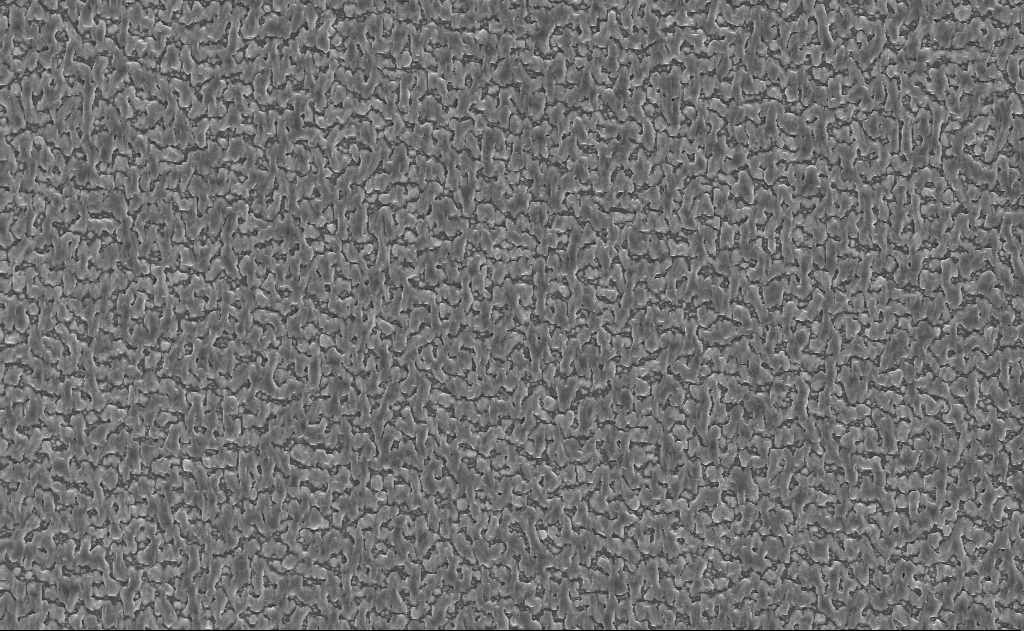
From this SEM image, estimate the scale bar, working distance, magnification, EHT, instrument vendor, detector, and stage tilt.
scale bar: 10000 nm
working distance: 14 mm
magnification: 5 K X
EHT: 10 kV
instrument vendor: Zeiss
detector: InLens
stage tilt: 0°